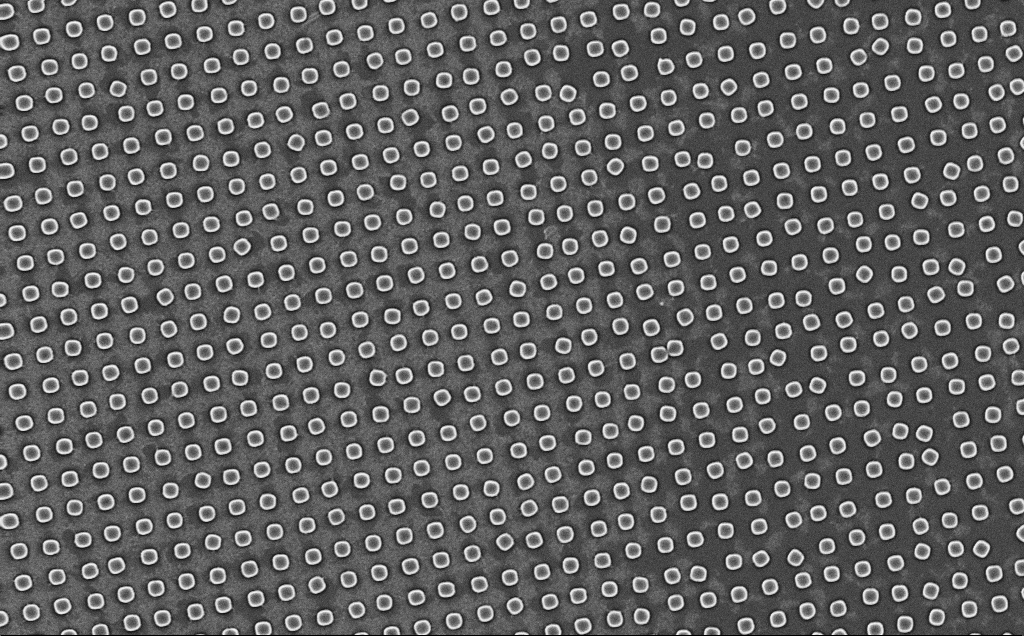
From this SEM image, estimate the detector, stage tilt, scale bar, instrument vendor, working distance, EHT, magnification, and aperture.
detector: InLens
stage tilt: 0°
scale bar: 2000 nm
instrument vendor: Zeiss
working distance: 7 mm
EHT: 5 kV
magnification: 7.78 K X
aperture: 30 µm